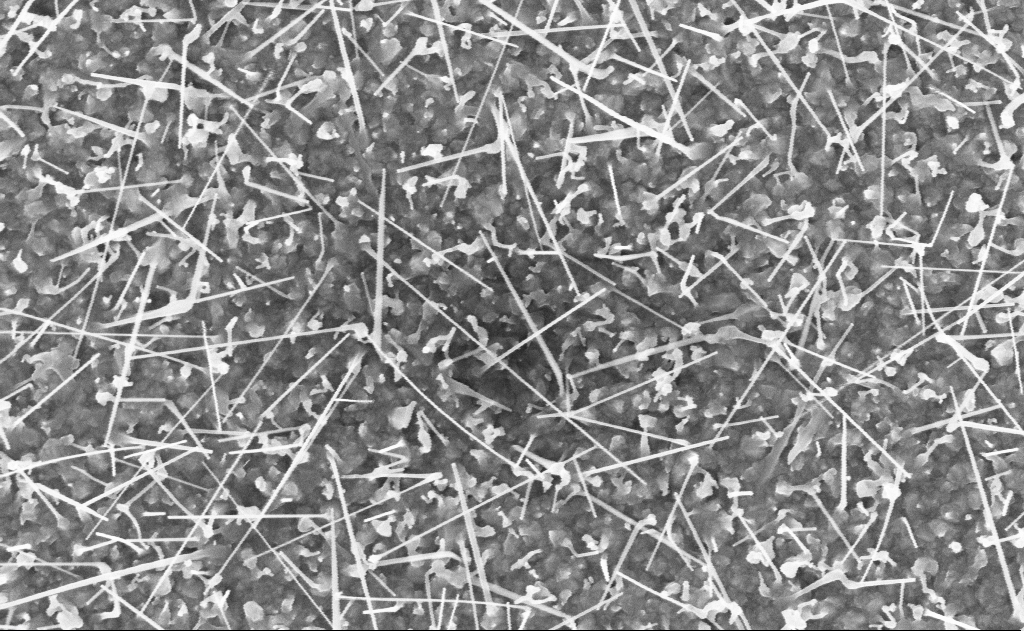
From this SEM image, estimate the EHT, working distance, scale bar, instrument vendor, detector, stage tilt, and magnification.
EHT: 10 kV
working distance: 20 mm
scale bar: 1000 nm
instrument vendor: Zeiss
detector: InLens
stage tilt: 0°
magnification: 40 K X